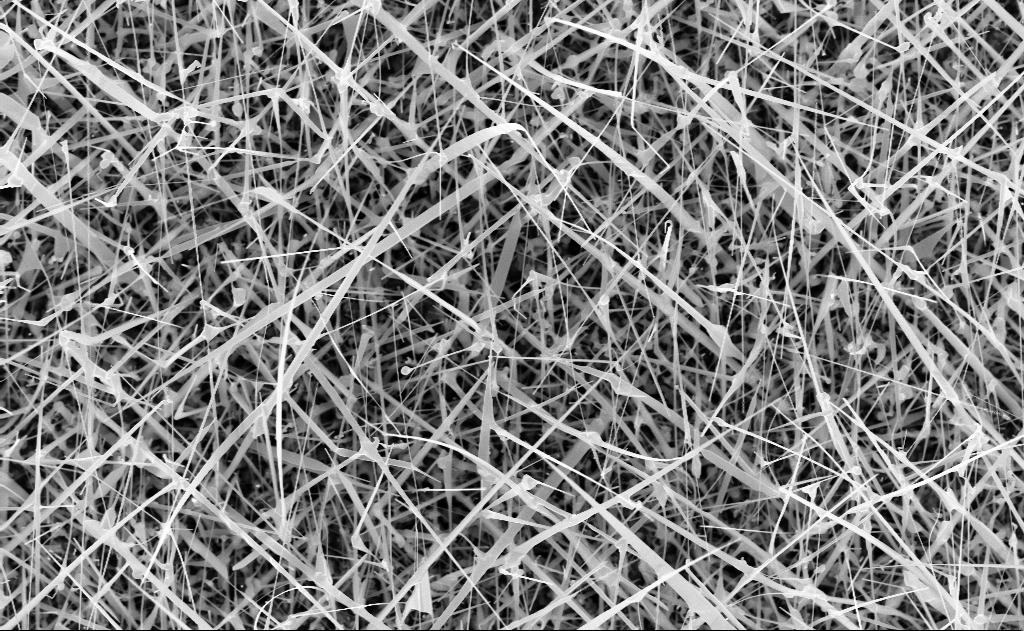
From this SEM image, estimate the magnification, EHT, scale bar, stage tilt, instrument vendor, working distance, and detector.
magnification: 20 K X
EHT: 10 kV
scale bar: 1000 nm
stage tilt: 0°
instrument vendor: Zeiss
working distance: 10 mm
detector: InLens